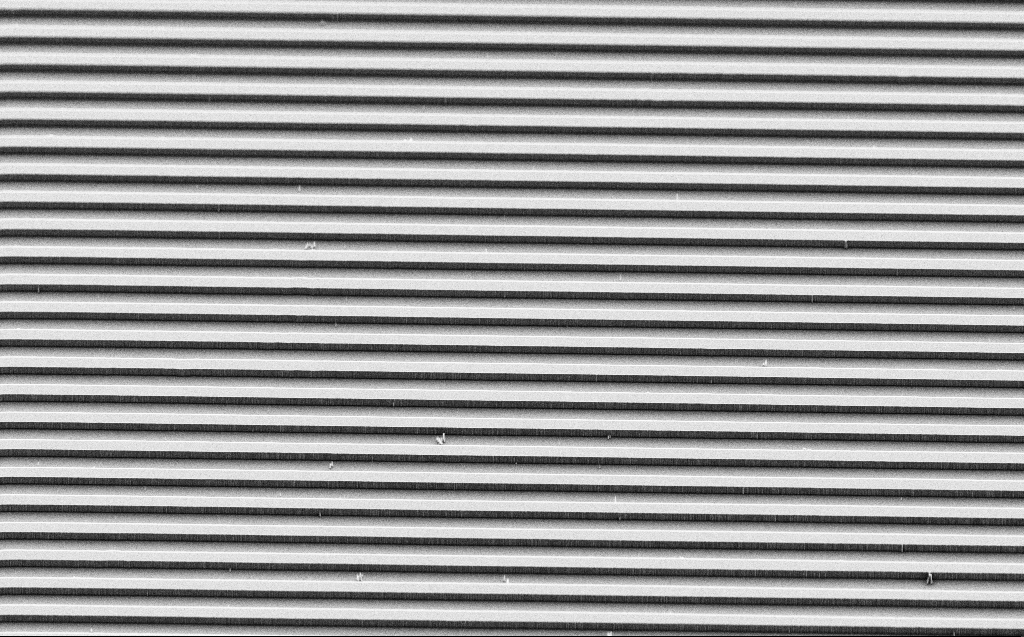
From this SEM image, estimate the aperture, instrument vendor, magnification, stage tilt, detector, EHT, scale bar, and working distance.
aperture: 30 µm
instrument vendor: Zeiss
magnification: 2.57 K X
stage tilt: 45°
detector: SE2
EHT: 3 kV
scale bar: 10000 nm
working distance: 9 mm